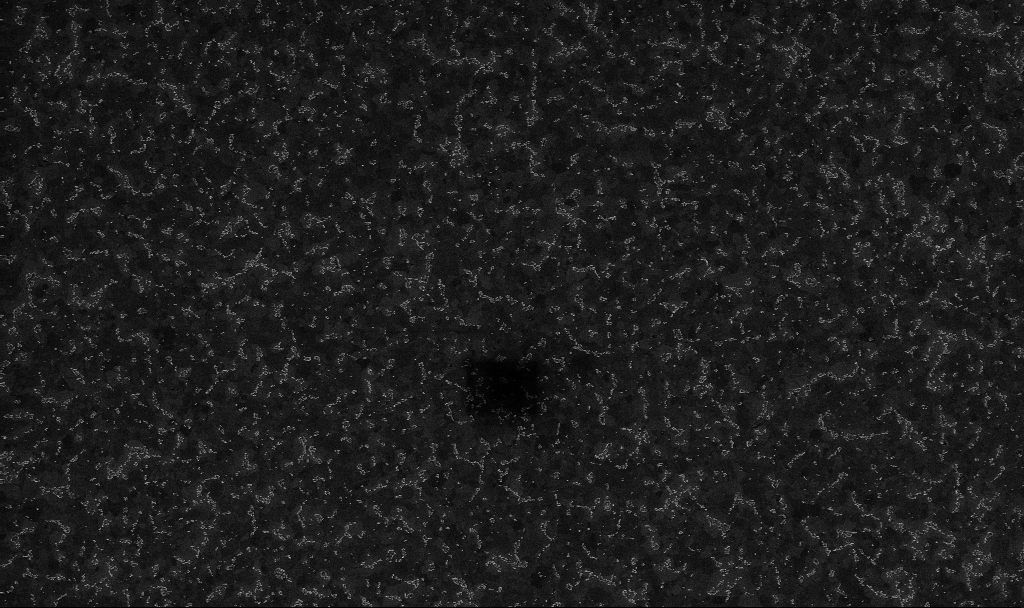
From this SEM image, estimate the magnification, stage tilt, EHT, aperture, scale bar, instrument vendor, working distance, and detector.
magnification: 20 K X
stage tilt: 0°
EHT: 10 kV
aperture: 30 µm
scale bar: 1000 nm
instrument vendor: Zeiss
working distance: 3.4 mm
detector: InLens